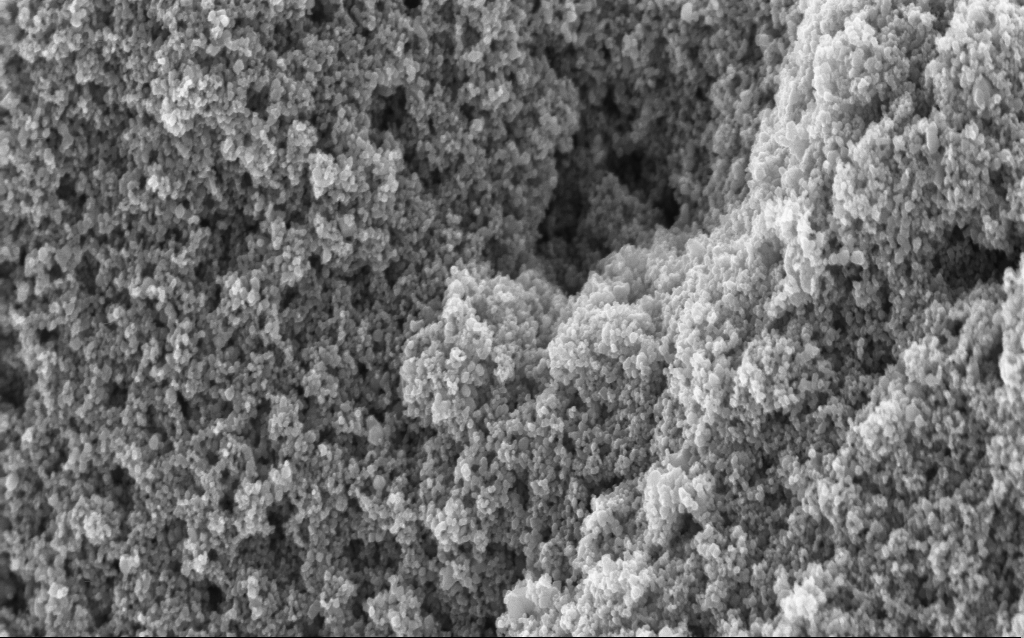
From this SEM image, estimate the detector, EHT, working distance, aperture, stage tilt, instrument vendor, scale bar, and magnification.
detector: InLens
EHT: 5 kV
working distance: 4.7 mm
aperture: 30 µm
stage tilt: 0°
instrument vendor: Zeiss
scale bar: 1000 nm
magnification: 68.32 K X